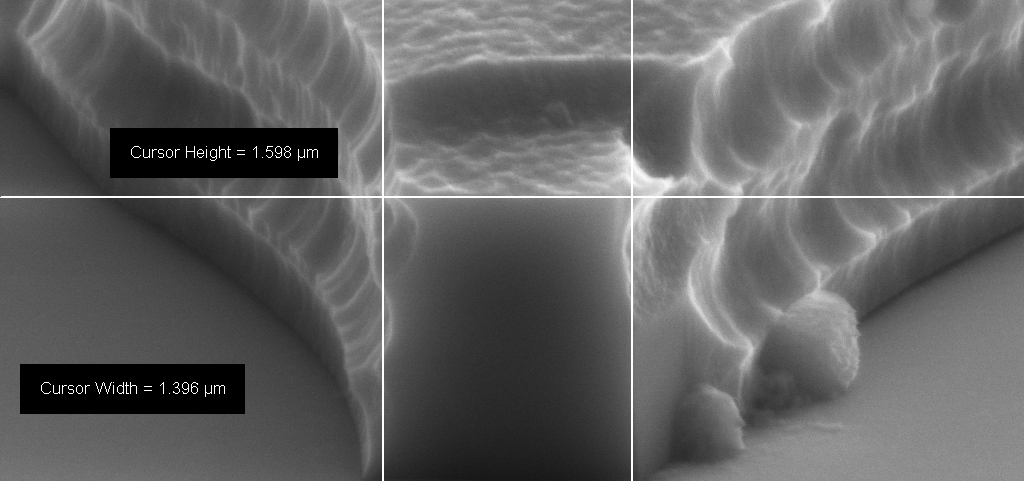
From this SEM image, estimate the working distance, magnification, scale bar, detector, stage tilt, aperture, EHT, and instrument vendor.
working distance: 12 mm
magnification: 65.5 K X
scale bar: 1000 nm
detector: SE2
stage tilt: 70°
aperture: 30 µm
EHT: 8 kV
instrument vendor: Zeiss